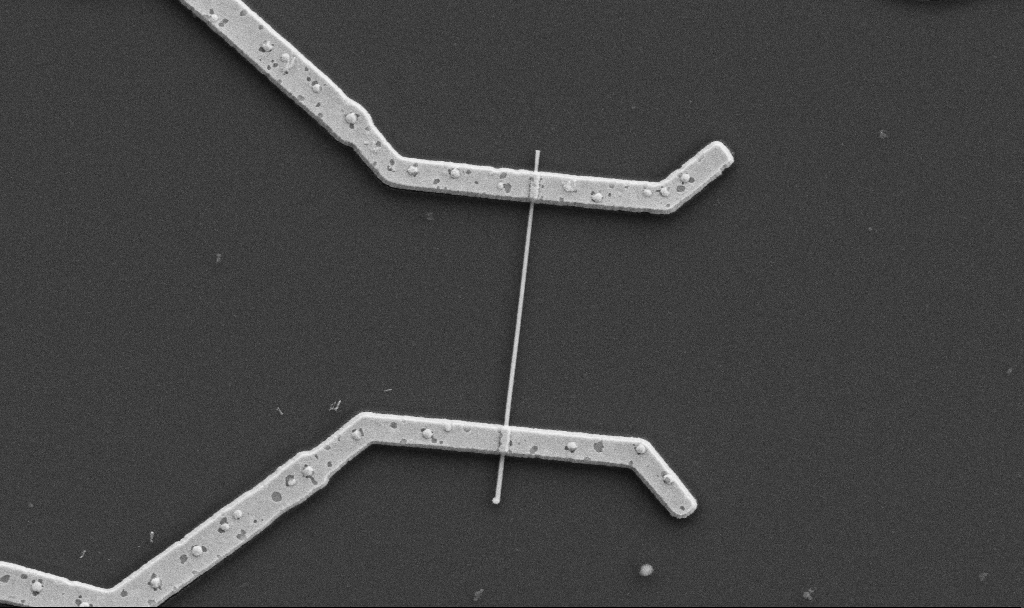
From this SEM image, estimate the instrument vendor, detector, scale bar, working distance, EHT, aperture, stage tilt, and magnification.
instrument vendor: Zeiss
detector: SE2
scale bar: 1000 nm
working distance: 10.6 mm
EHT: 5 kV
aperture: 30 µm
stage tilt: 0°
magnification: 20 K X